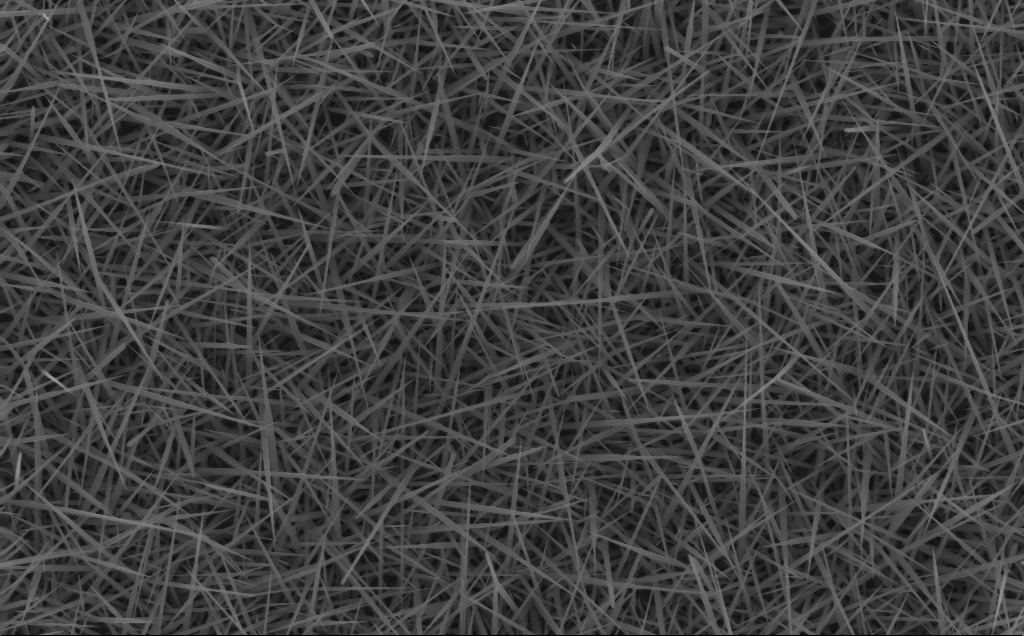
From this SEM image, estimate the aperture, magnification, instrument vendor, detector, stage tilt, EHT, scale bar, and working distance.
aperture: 30 µm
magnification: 20 K X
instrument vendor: Zeiss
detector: InLens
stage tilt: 0°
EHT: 10 kV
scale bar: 2000 nm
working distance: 4 mm